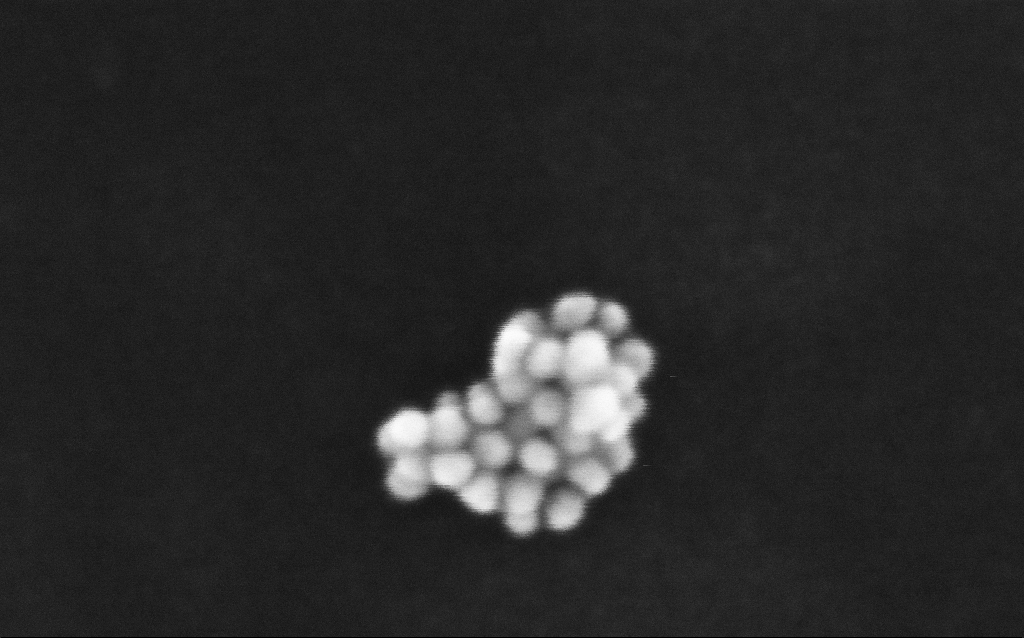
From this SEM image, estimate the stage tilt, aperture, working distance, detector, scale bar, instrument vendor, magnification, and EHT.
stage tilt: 0°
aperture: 30 µm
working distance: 3 mm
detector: InLens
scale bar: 20 nm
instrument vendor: Zeiss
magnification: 965.88 K X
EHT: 6 kV